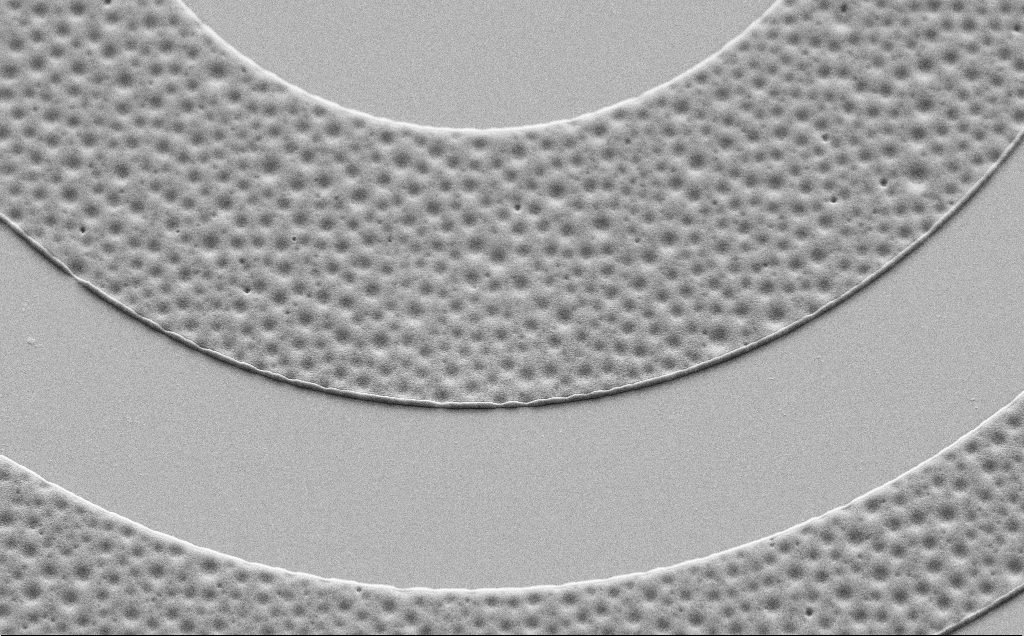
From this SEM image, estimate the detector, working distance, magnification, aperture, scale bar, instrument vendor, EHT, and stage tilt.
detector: SE2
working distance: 7 mm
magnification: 10.16 K X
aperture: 30 µm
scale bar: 2000 nm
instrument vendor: Zeiss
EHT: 5 kV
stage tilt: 45°